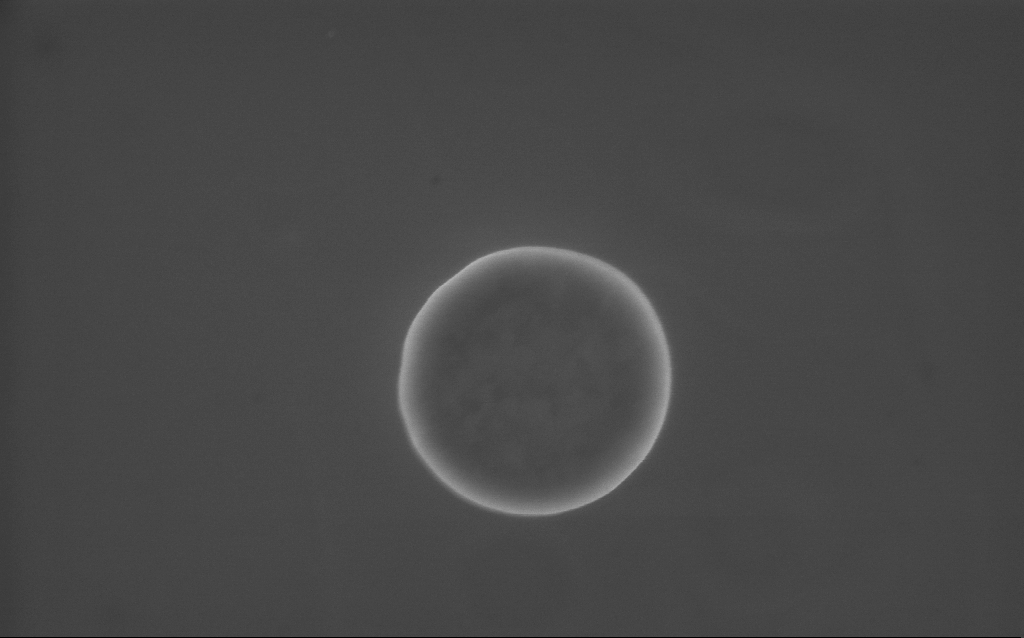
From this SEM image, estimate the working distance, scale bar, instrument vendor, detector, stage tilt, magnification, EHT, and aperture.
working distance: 4 mm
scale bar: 1000 nm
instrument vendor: Zeiss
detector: InLens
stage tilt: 0°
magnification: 70 K X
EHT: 10 kV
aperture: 30 µm